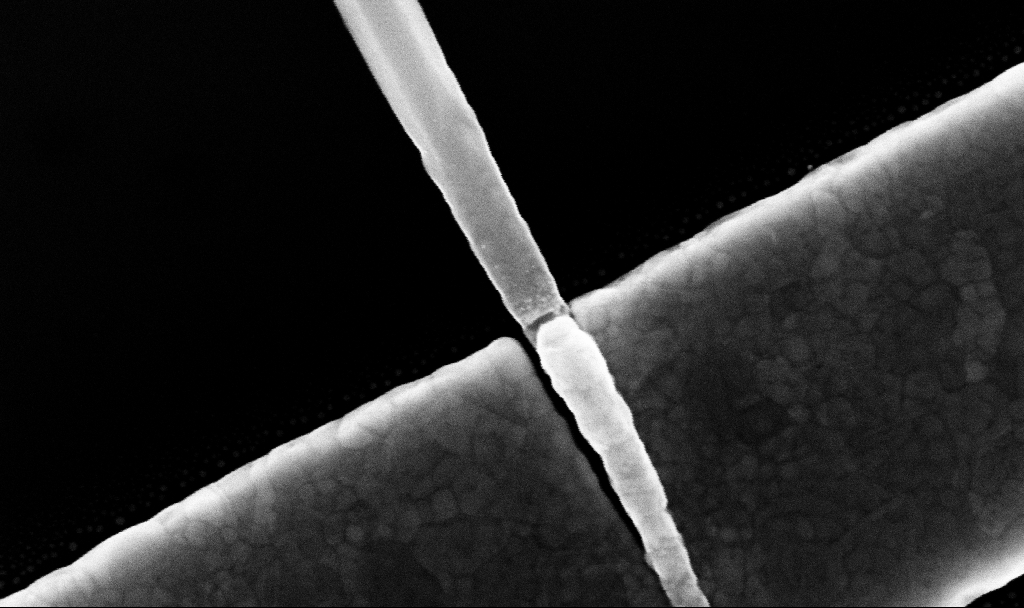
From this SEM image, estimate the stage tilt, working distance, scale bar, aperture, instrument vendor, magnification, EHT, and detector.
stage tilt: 0°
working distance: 7.7 mm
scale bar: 200 nm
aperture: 30 µm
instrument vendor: Zeiss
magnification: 189.51 K X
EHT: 10 kV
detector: InLens